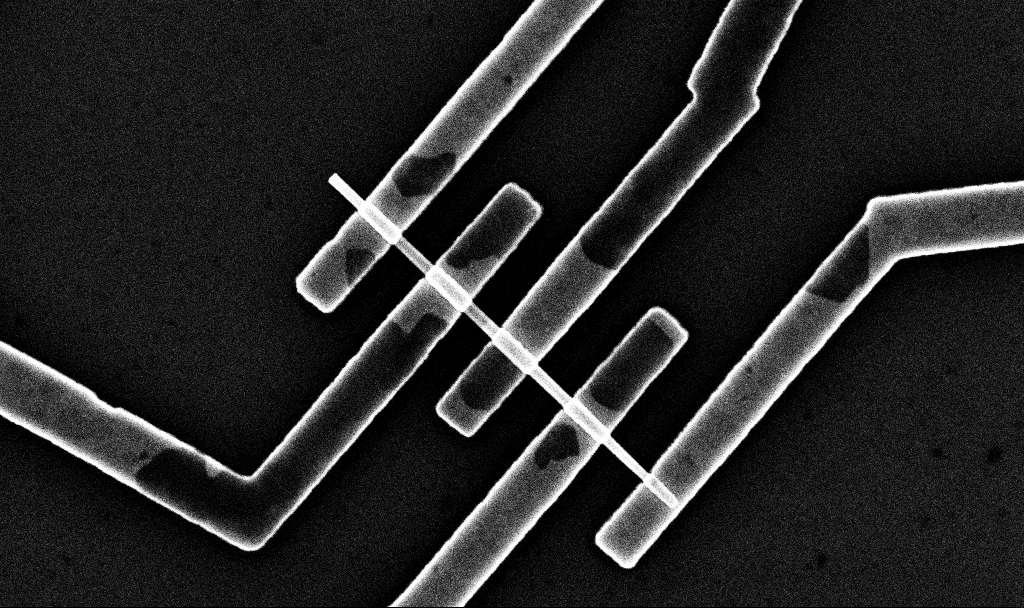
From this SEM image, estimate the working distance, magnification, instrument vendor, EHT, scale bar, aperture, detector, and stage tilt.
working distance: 6.7 mm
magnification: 32.22 K X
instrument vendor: Zeiss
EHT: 10 kV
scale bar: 2000 nm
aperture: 30 µm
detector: InLens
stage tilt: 0°